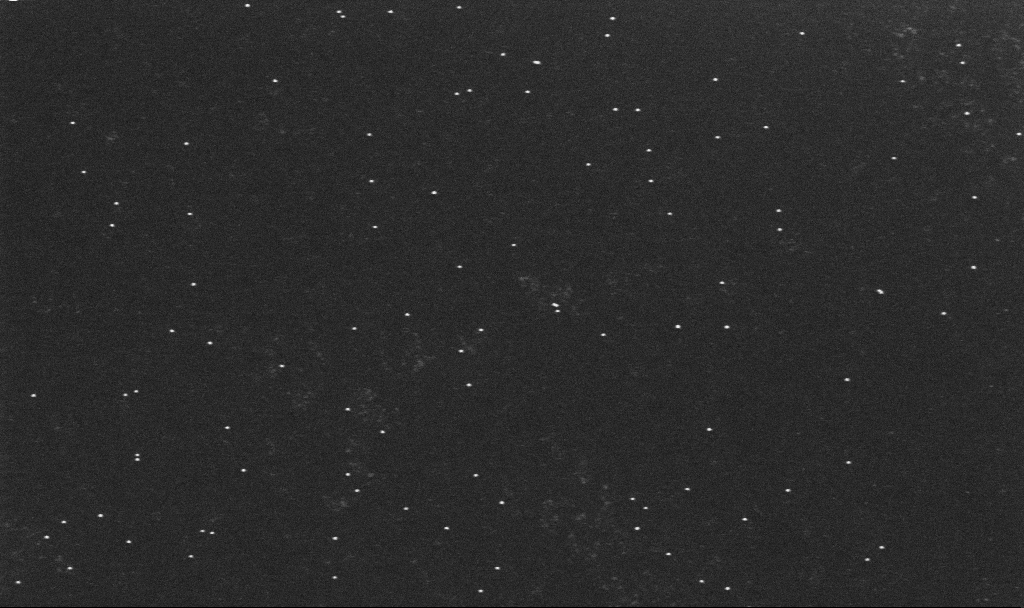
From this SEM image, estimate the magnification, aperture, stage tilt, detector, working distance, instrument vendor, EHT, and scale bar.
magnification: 100 K X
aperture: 30 µm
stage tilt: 0°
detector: InLens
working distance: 3.2 mm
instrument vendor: Zeiss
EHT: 10 kV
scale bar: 200 nm